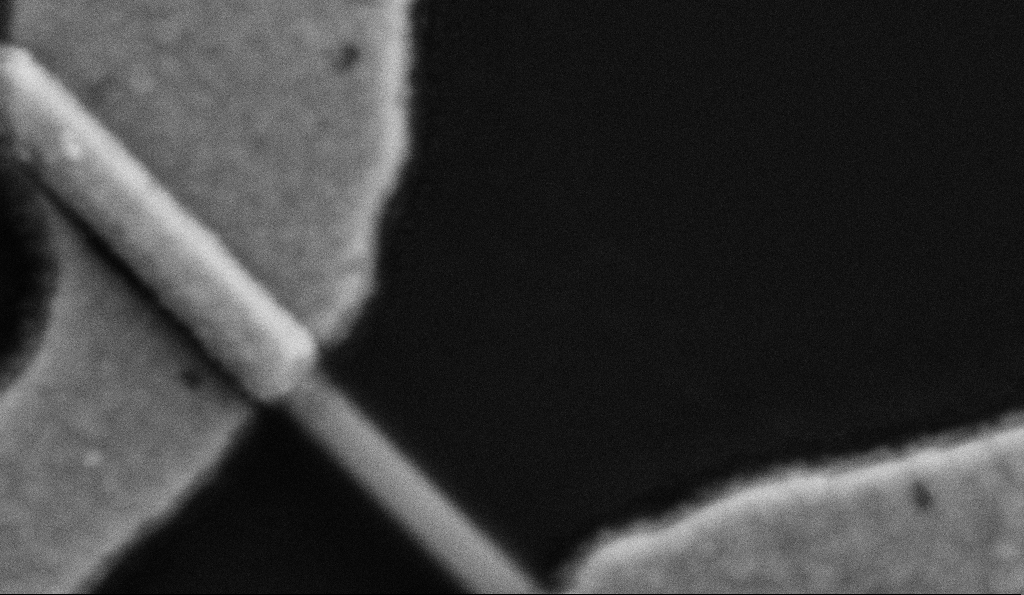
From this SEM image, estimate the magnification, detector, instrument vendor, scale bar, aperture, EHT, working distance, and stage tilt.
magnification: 200 K X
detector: SE2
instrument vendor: Zeiss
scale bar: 200 nm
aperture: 30 µm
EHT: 5 kV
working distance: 8.5 mm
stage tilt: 0°